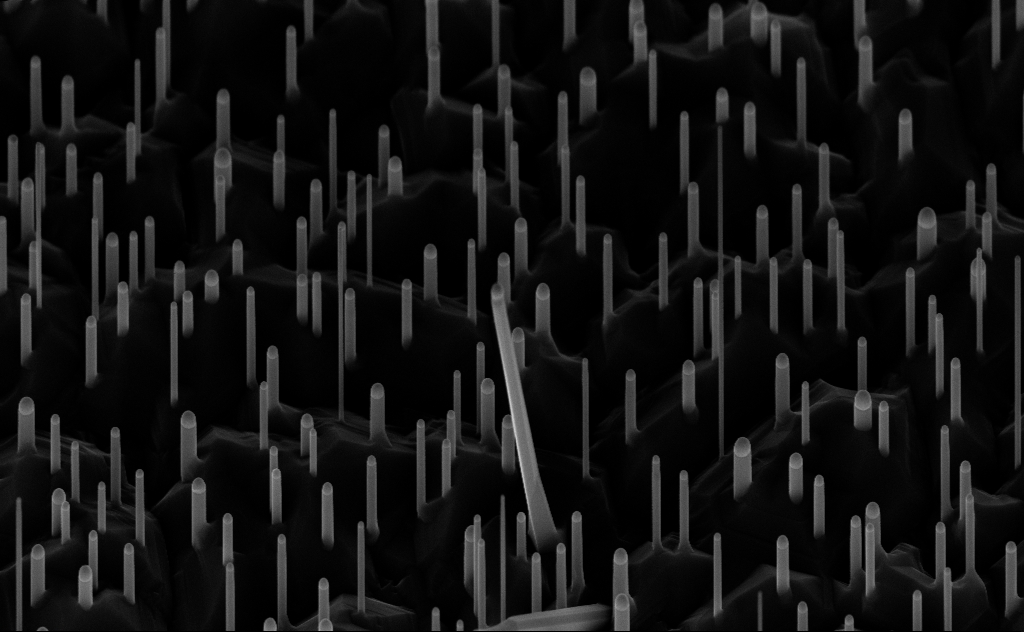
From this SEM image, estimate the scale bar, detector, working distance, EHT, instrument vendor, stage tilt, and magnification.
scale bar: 1000 nm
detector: InLens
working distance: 7 mm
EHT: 10 kV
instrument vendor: Zeiss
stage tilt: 45°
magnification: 40 K X